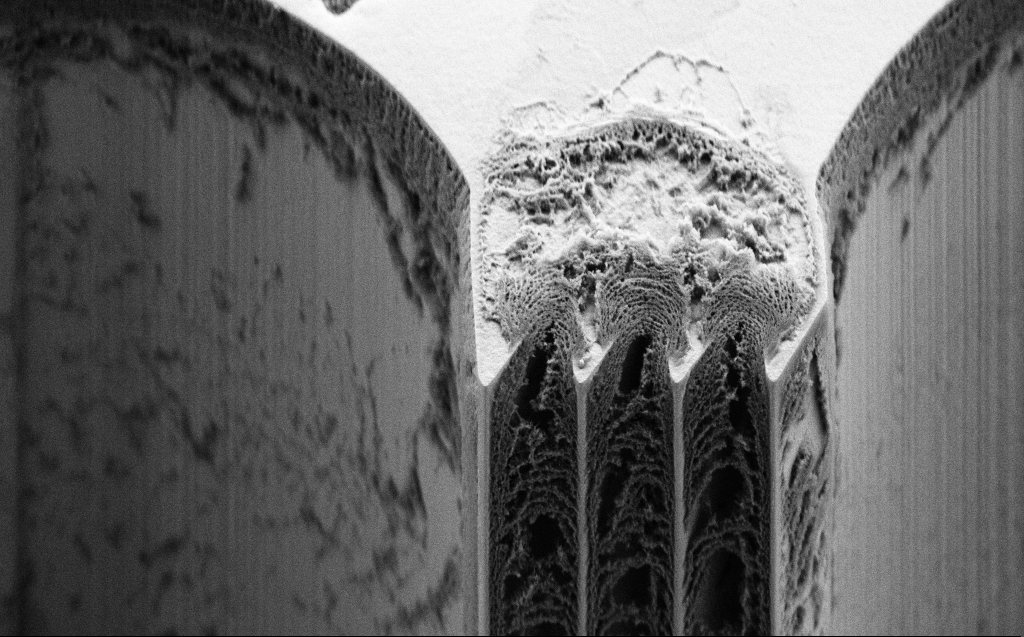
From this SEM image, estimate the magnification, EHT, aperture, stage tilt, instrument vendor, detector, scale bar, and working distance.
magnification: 3.13 K X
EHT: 5 kV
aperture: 30 µm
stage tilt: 45°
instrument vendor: Zeiss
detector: SE2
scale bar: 10000 nm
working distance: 7 mm